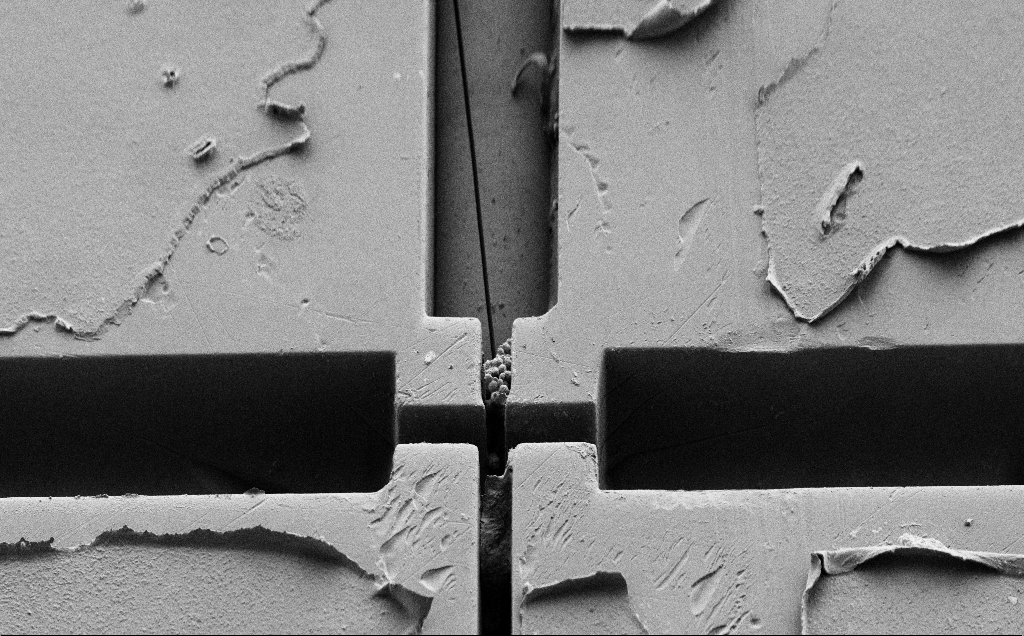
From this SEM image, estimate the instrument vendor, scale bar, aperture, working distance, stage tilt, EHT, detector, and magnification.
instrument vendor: Zeiss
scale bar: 20000 nm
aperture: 30 µm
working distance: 5 mm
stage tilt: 45°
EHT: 1 kV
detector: SE2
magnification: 0.942 K X